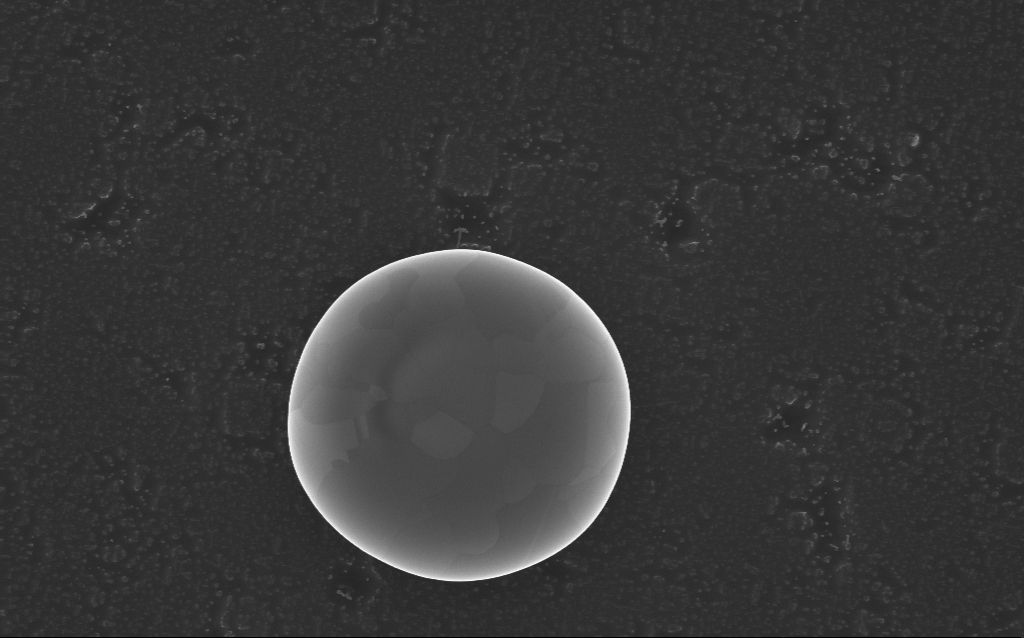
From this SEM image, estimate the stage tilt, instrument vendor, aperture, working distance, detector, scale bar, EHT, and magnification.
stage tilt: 0°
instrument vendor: Zeiss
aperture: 30 µm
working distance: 5 mm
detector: InLens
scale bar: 1000 nm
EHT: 10 kV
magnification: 49.55 K X